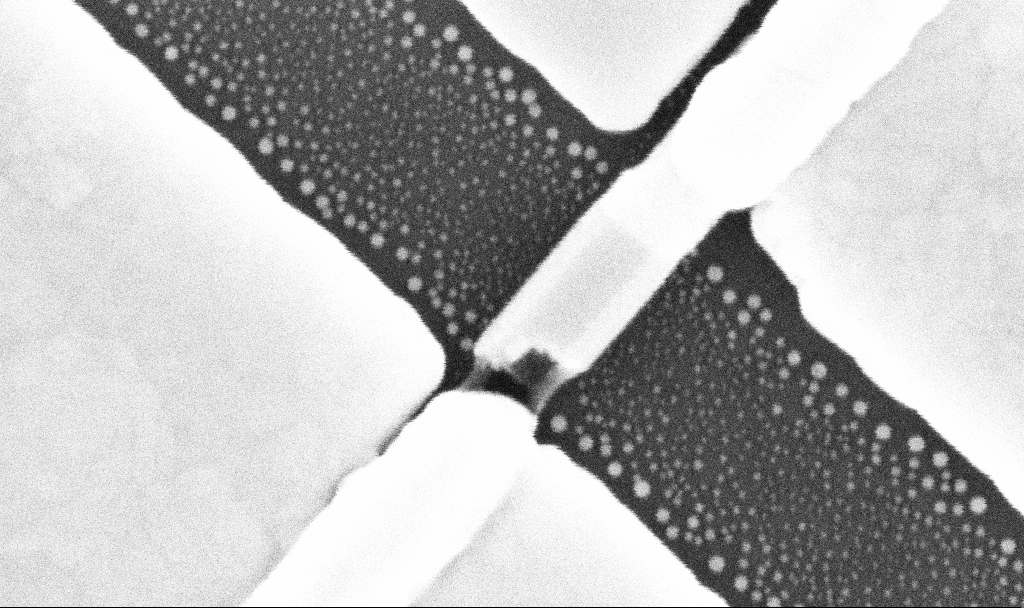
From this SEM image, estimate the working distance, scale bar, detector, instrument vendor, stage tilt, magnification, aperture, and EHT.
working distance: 7.7 mm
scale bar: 200 nm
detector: InLens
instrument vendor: Zeiss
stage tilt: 0°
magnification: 335.01 K X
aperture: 30 µm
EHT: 10 kV